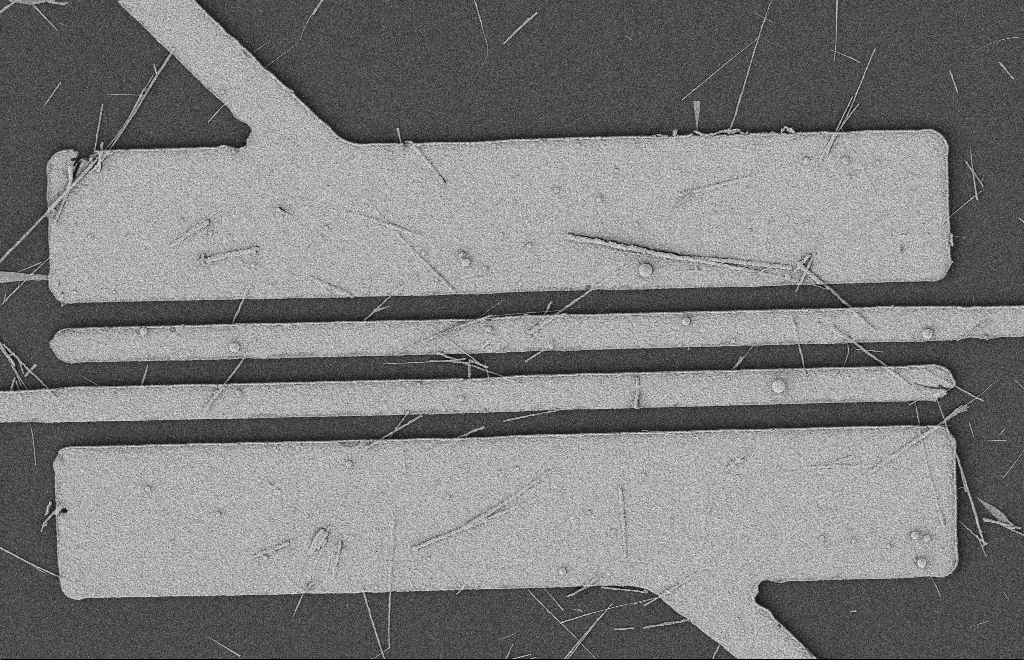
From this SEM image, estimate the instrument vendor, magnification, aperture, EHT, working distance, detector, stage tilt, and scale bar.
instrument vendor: Zeiss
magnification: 5.39 K X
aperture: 20 µm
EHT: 2 kV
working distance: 12 mm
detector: SE2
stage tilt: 0°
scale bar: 2000 nm